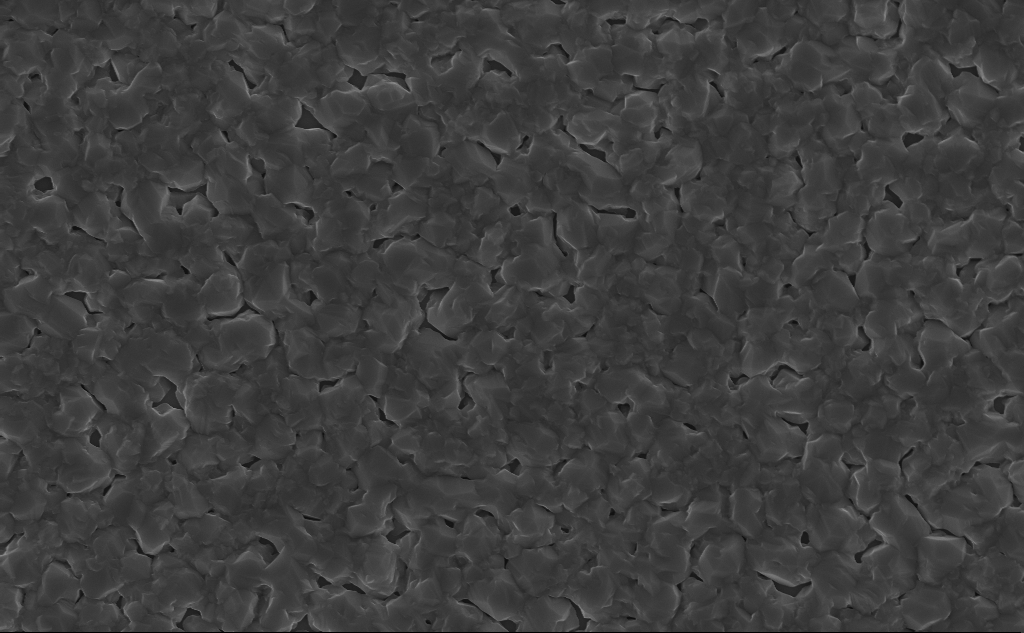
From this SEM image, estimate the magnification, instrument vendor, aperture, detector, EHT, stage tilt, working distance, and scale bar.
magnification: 18.47 K X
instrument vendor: Zeiss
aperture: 30 µm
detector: InLens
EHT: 10 kV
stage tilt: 0°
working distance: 6 mm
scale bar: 1000 nm